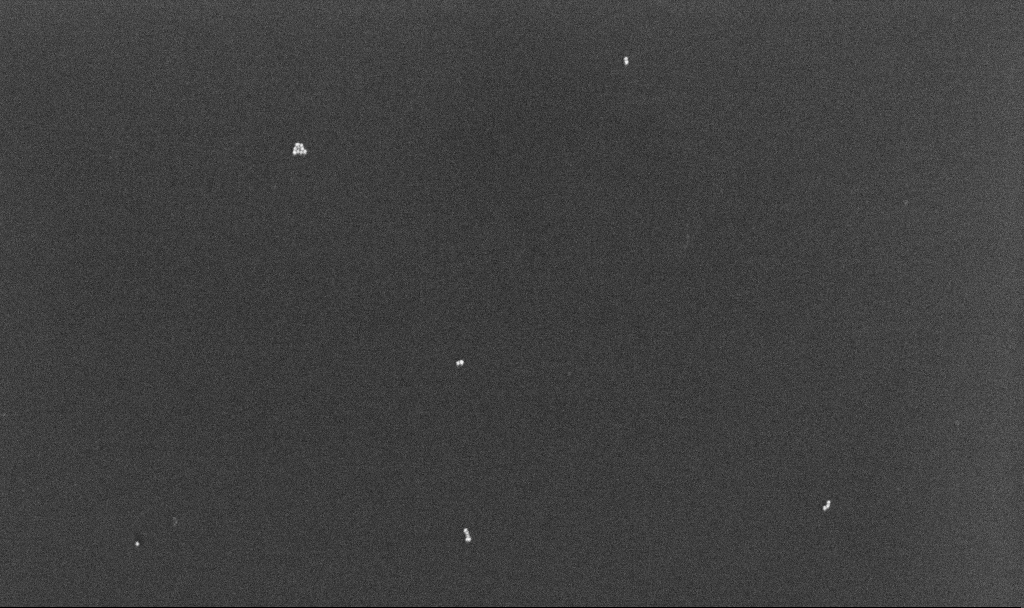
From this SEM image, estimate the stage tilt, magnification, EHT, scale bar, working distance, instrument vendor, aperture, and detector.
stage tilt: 0°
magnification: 81.14 K X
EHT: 10 kV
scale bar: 200 nm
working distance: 4.3 mm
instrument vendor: Zeiss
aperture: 30 µm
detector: InLens